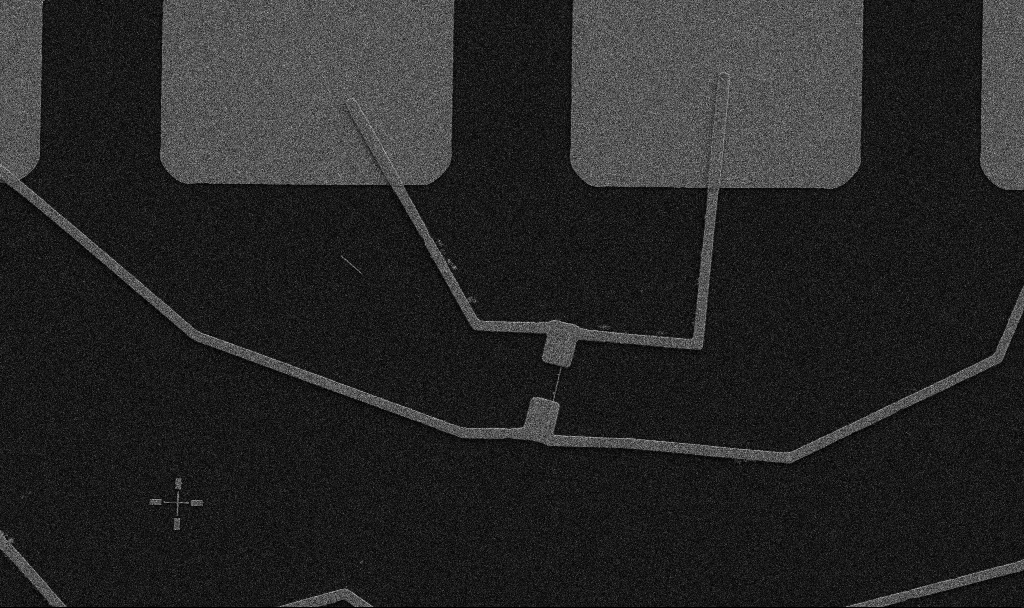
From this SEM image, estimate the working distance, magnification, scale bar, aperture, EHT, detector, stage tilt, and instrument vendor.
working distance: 10.7 mm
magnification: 5 K X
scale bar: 10000 nm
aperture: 30 µm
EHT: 5 kV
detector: SE2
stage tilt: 0°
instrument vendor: Zeiss